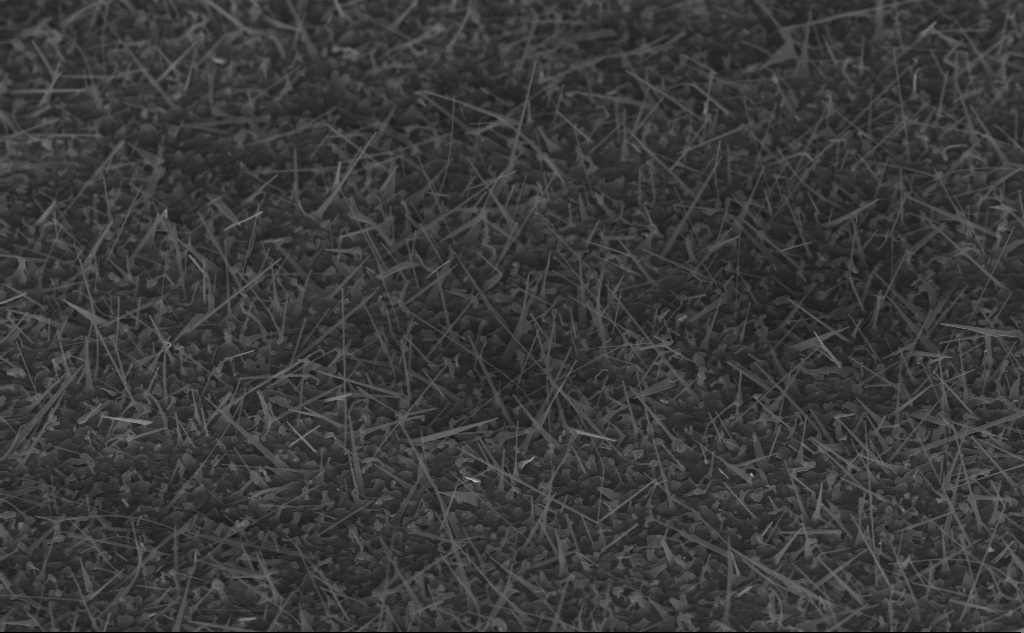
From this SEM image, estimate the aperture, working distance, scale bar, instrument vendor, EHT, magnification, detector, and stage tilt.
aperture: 30 µm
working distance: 8 mm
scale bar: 1000 nm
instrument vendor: Zeiss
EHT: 10 kV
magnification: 20 K X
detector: InLens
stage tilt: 45°